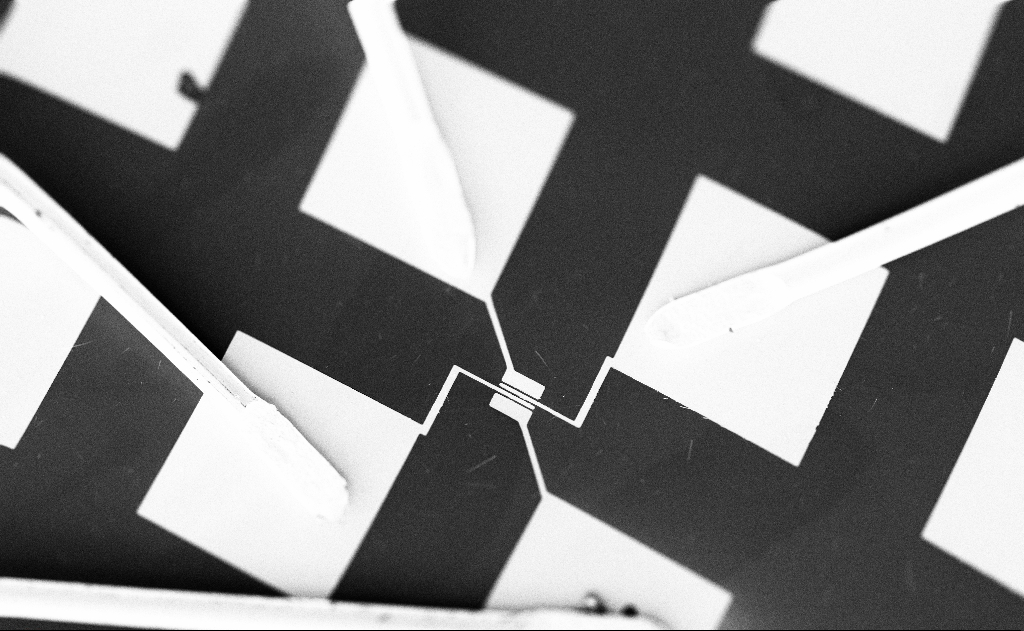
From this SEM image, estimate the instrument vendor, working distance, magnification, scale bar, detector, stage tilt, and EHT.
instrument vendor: Zeiss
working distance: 20 mm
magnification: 0.508 K X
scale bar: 100000 nm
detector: SE2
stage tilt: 0°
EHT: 5 kV